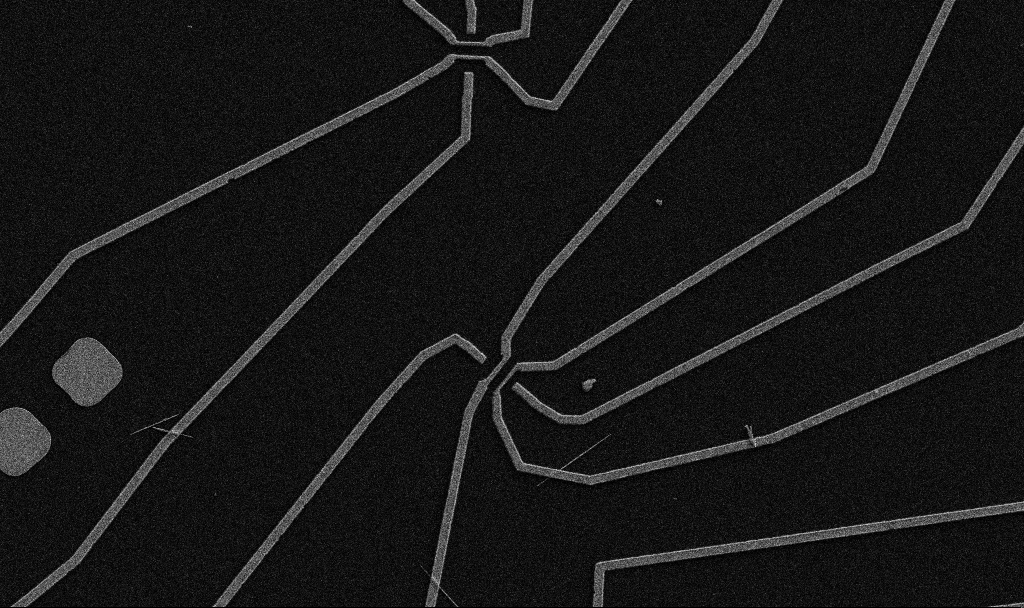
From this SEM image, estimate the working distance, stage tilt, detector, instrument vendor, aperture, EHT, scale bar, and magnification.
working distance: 10.7 mm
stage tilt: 0°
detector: SE2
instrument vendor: Zeiss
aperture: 30 µm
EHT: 5 kV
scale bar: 10000 nm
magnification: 5 K X